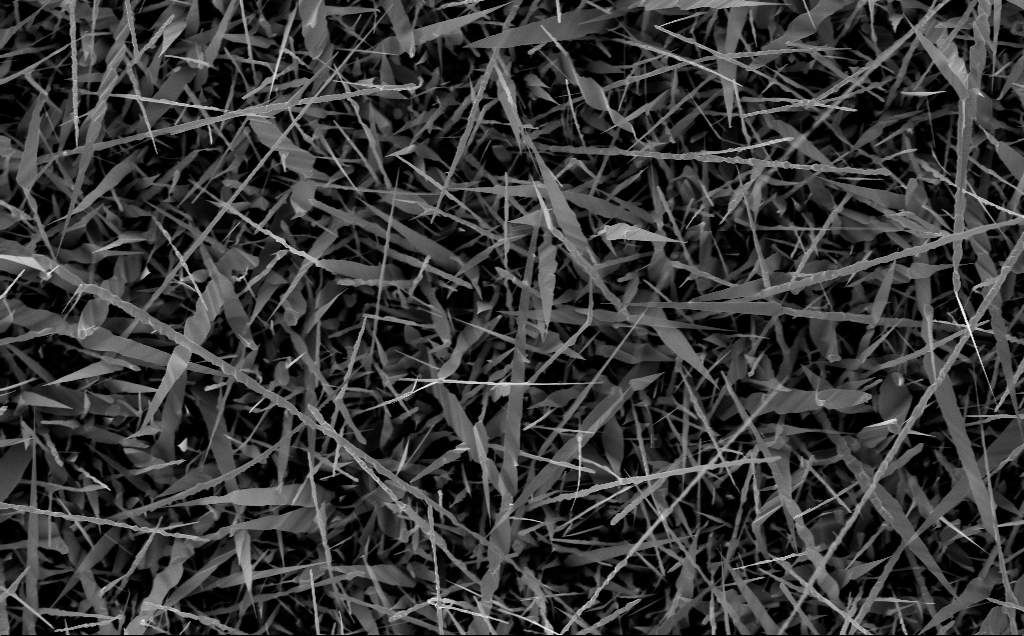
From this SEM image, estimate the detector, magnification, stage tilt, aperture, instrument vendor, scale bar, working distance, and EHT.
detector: InLens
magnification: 10 K X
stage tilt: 0°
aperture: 30 µm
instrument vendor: Zeiss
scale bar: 2000 nm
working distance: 6 mm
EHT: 10 kV